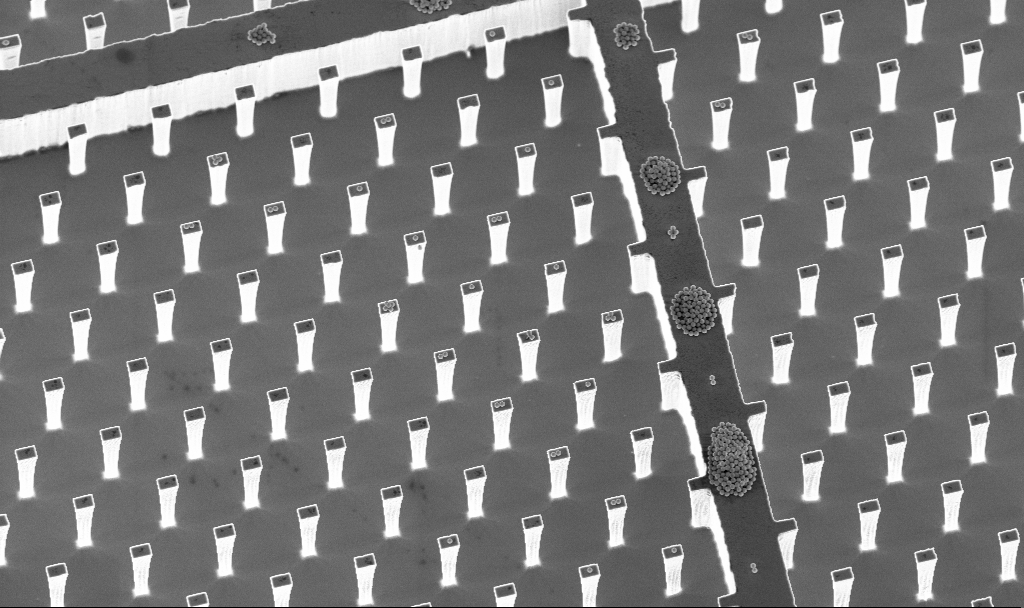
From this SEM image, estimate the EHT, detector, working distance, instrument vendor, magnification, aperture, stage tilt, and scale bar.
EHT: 5 kV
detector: InLens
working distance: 4.7 mm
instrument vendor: Zeiss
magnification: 2.64 K X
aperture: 30 µm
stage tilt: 20°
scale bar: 10000 nm